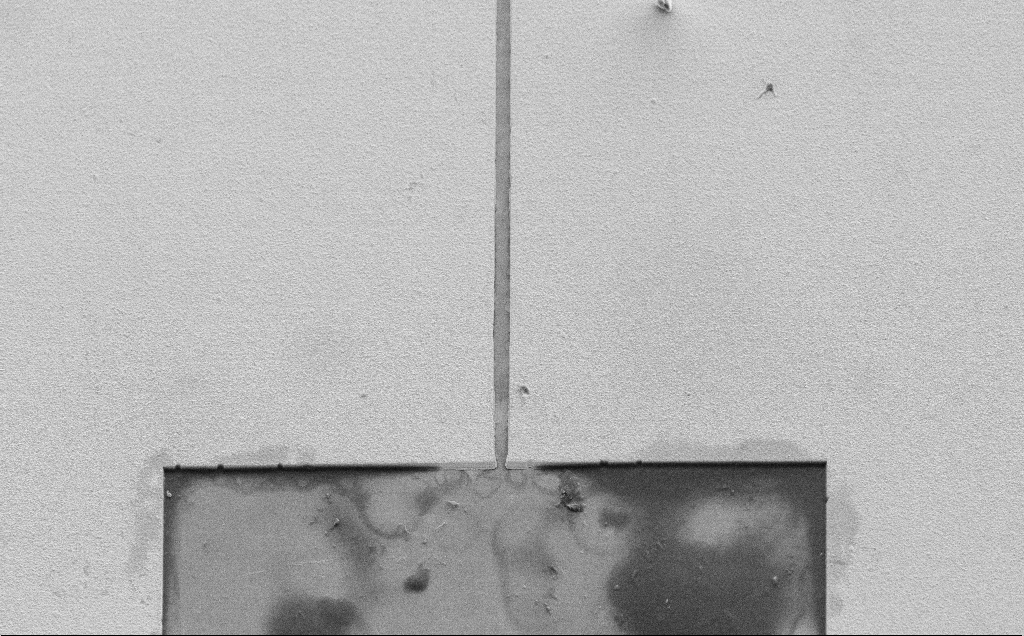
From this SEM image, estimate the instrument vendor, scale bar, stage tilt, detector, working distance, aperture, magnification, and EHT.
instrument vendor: Zeiss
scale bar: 100000 nm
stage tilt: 45°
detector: SE2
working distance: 11 mm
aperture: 30 µm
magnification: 0.609 K X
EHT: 10 kV